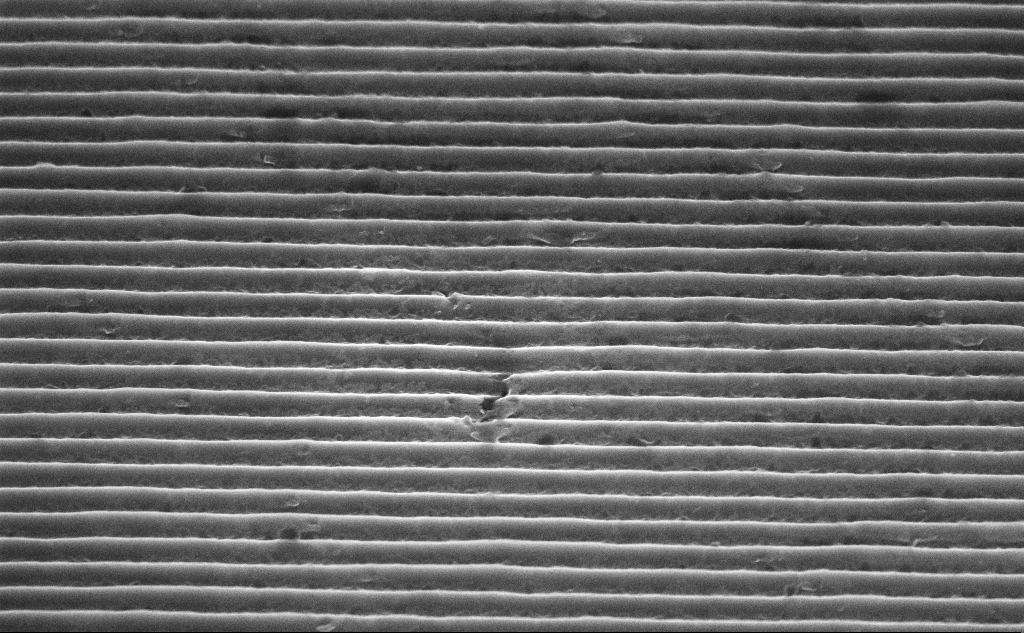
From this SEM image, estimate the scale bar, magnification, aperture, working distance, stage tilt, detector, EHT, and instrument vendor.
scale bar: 1000 nm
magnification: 43.87 K X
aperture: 30 µm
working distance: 7.4 mm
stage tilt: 45°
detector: InLens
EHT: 5 kV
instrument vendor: Zeiss